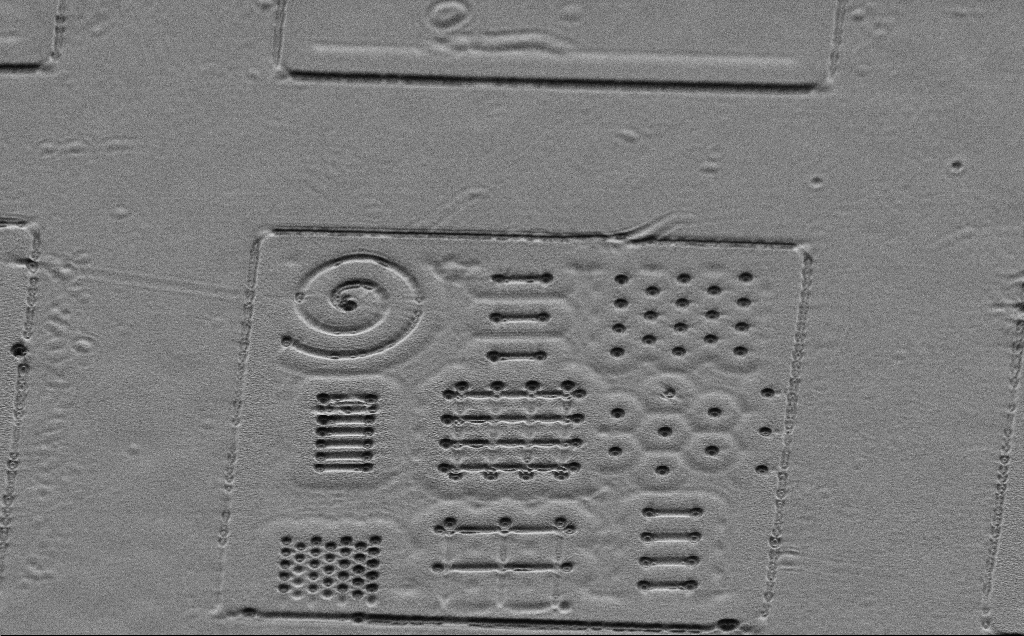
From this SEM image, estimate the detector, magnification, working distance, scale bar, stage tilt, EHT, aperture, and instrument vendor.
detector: SE2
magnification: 2.02 K X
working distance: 6 mm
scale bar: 10000 nm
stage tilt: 45°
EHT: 1 kV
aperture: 30 µm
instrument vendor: Zeiss